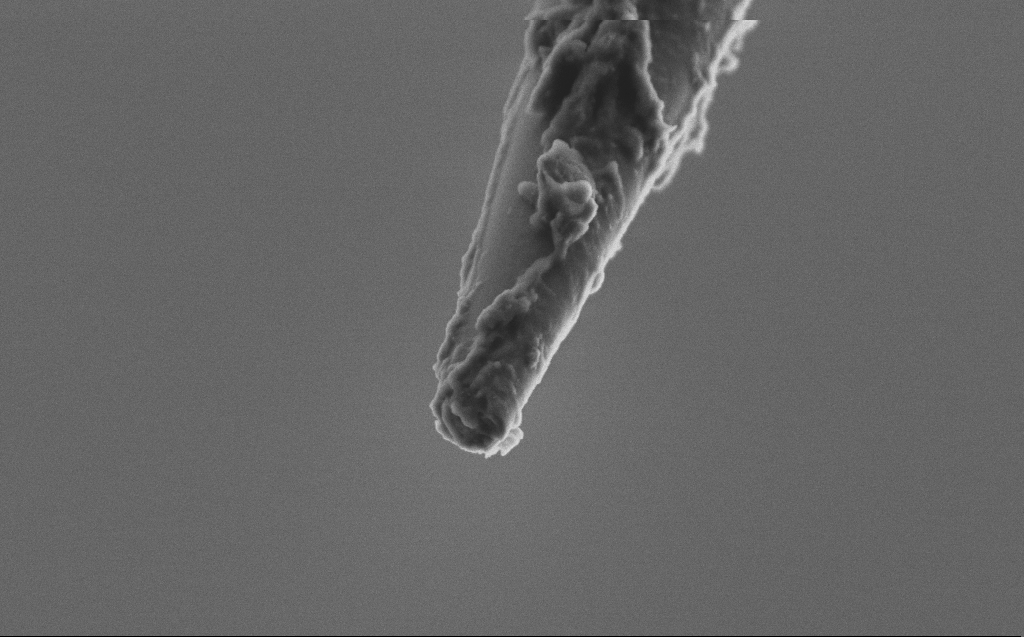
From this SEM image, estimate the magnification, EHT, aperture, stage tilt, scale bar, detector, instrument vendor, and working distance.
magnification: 50 K X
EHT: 2 kV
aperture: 30 µm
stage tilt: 45°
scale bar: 1000 nm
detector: SE2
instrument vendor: Zeiss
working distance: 3 mm